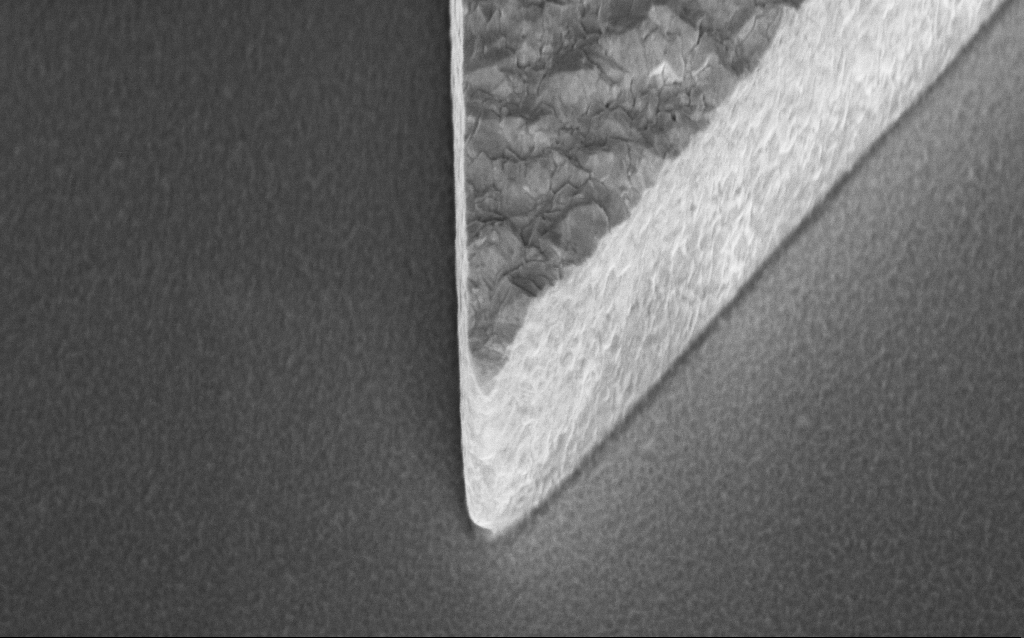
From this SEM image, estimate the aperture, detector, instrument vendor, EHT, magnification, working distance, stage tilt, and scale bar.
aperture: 30 µm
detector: InLens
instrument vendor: Zeiss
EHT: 3 kV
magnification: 60.8 K X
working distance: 6.4 mm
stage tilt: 45°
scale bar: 1000 nm